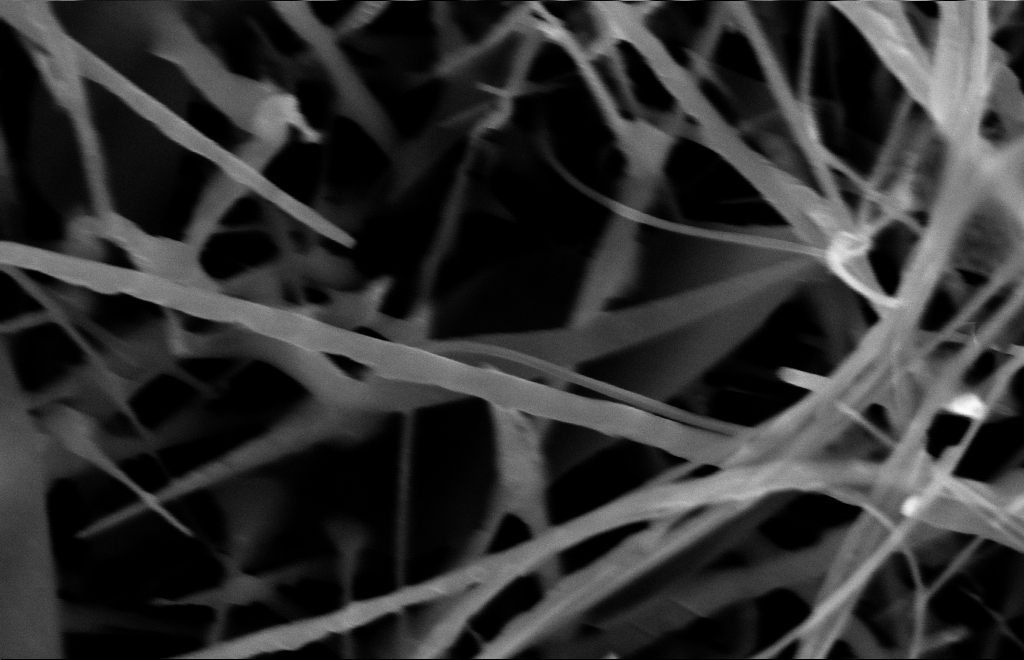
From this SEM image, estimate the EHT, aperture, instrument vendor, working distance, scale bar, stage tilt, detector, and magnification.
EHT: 10 kV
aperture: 30 µm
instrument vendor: Zeiss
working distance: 14 mm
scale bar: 100 nm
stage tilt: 0°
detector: InLens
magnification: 80 K X